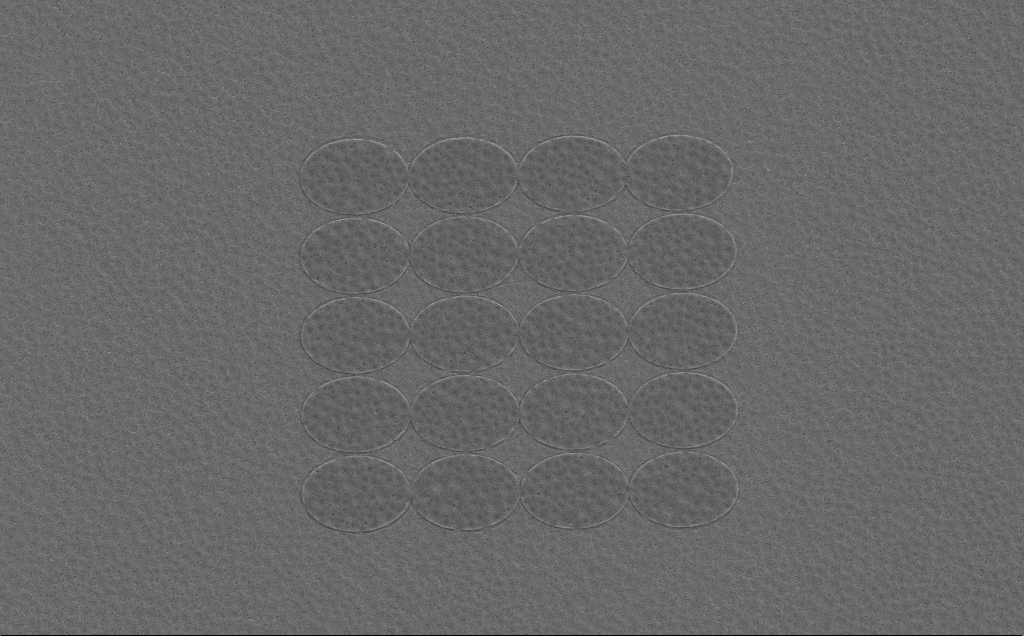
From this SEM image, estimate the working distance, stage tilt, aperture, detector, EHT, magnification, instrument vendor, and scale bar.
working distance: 6 mm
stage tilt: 0°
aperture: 30 µm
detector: SE2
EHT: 5 kV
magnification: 4.03 K X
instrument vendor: Zeiss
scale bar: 10000 nm